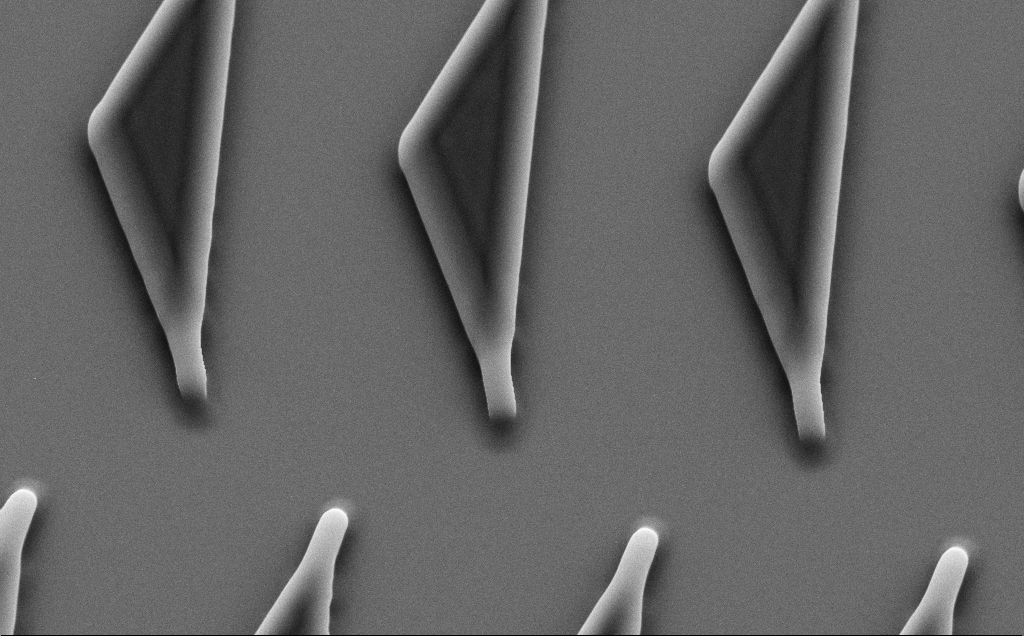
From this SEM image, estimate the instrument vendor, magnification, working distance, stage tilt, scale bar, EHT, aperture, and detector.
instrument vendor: Zeiss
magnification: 6.74 K X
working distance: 8 mm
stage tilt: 35°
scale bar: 10000 nm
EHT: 10 kV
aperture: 30 µm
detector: SE2